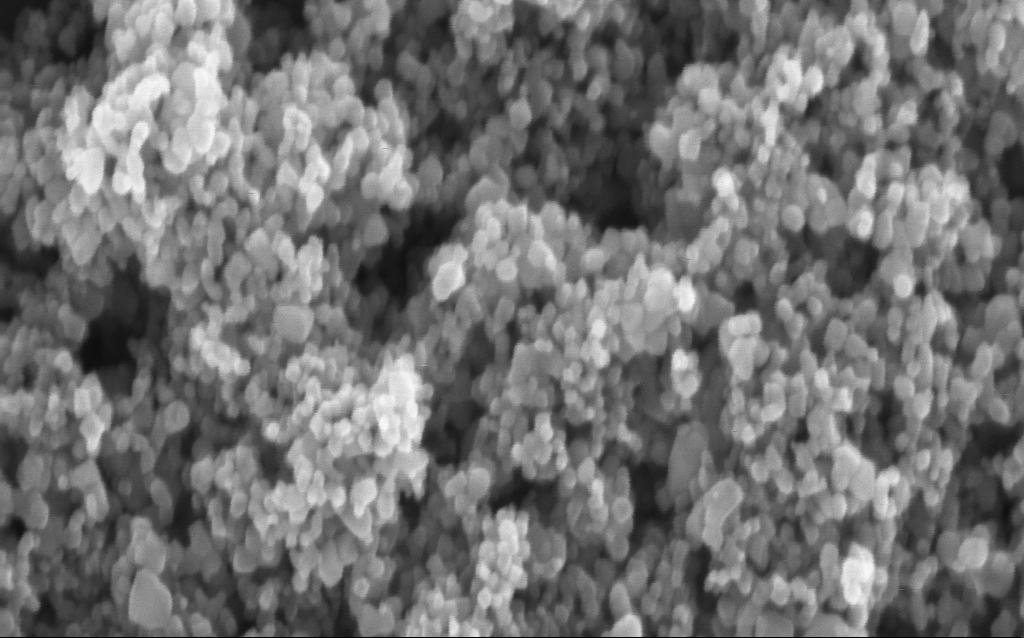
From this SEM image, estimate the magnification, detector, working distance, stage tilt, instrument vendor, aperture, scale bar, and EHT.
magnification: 225.07 K X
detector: InLens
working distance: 4.7 mm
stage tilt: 0°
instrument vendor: Zeiss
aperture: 30 µm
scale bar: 200 nm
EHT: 5 kV